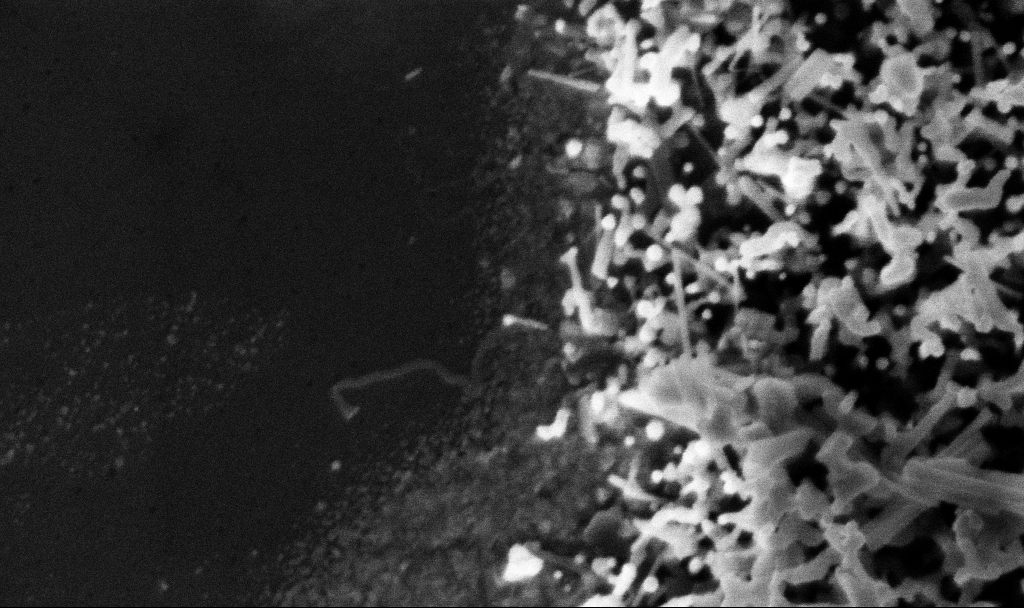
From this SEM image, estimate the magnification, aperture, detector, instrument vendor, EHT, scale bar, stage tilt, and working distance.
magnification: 150.37 K X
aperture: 30 µm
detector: InLens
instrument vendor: Zeiss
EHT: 3 kV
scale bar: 200 nm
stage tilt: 0°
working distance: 3.1 mm